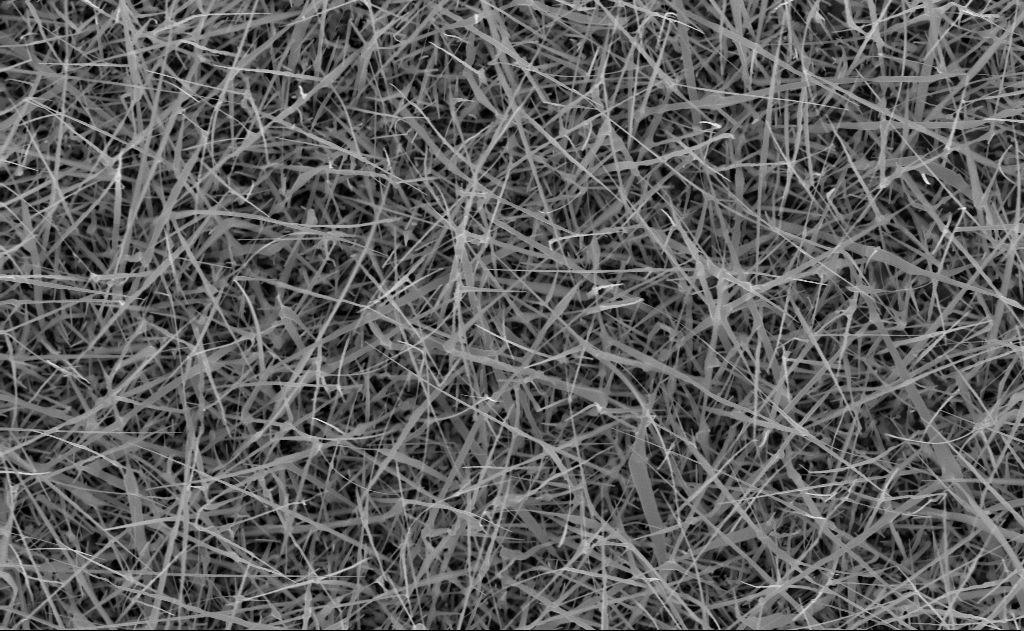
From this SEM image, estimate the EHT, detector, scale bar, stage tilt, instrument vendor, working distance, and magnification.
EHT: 10 kV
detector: InLens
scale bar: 2000 nm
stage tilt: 0°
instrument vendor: Zeiss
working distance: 10 mm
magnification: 20 K X